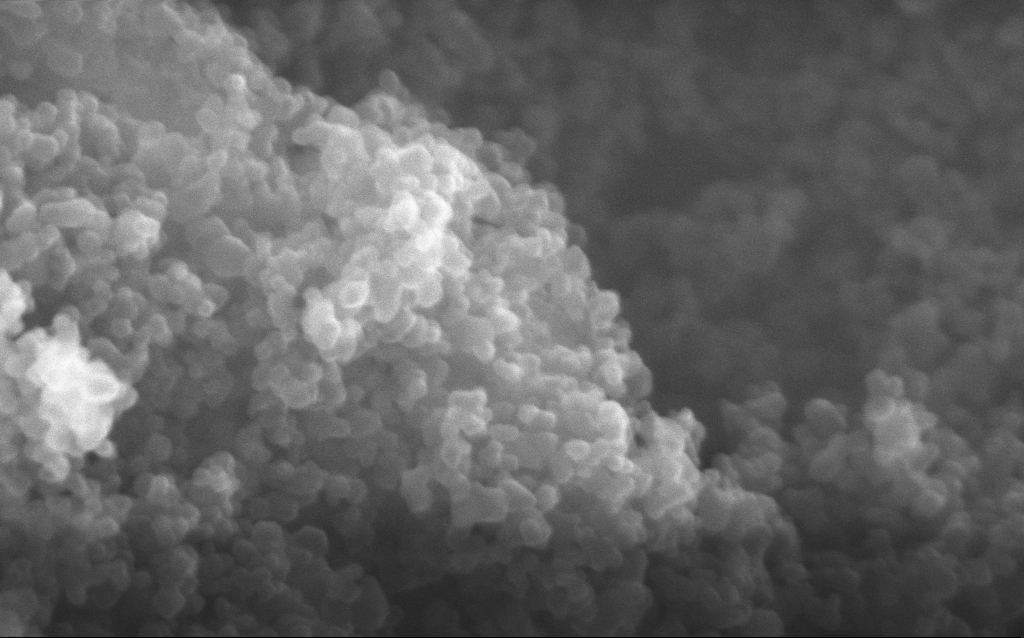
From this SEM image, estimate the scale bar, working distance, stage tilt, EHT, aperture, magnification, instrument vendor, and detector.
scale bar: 100 nm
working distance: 2.7 mm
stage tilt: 0°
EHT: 10 kV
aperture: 30 µm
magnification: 348.1 K X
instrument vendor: Zeiss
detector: InLens